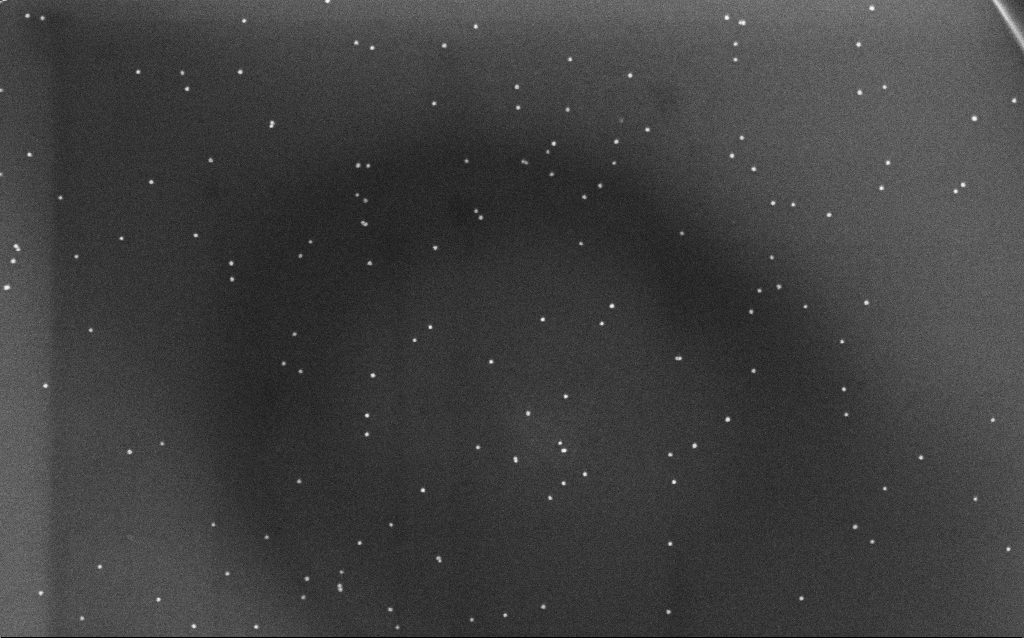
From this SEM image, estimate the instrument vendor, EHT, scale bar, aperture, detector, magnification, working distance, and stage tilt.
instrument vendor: Zeiss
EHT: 10 kV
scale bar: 200 nm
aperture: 30 µm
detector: InLens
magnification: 100 K X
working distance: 6.4 mm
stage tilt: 0°